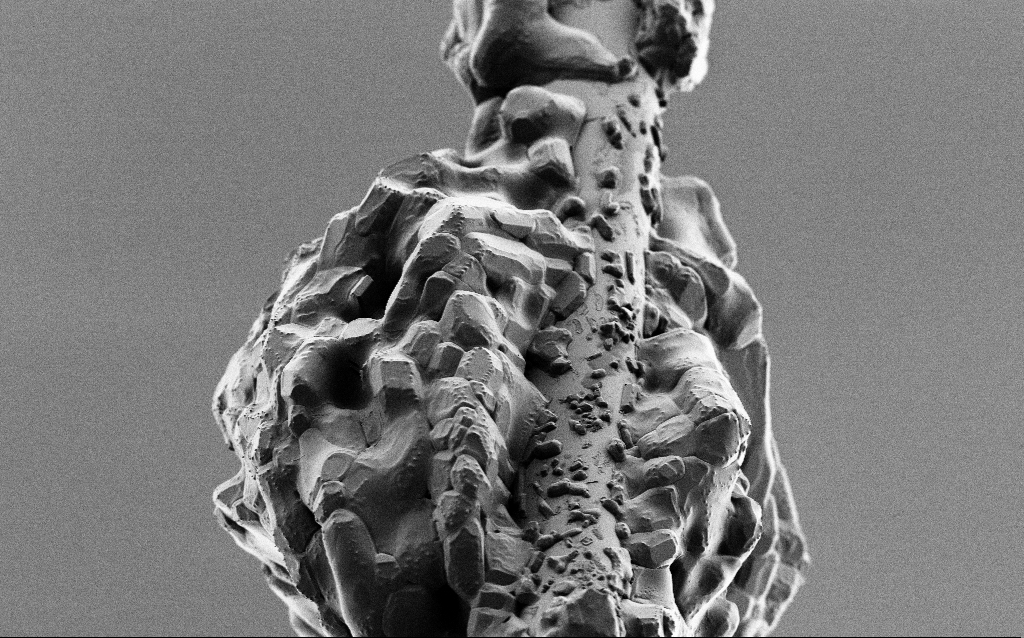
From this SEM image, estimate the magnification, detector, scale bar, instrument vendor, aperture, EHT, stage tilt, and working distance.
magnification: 2.5 K X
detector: SE2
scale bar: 10000 nm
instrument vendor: Zeiss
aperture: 30 µm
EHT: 1 kV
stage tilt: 45°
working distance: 6.5 mm